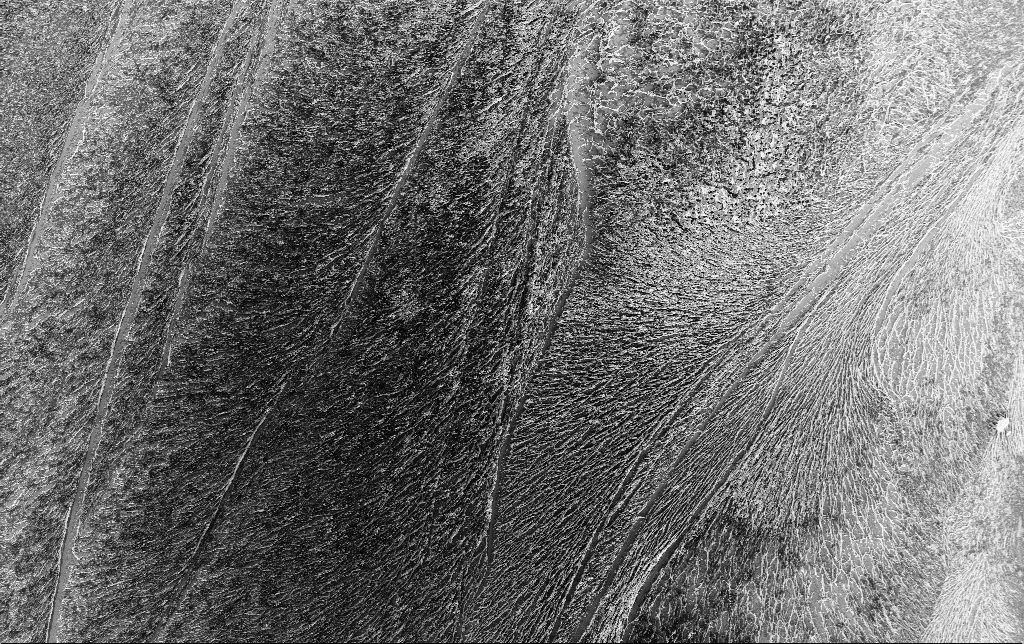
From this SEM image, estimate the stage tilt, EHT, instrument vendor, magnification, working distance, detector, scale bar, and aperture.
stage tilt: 0°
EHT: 3 kV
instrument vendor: Zeiss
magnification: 0.463 K X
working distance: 3.2 mm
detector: InLens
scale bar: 100000 nm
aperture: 30 µm